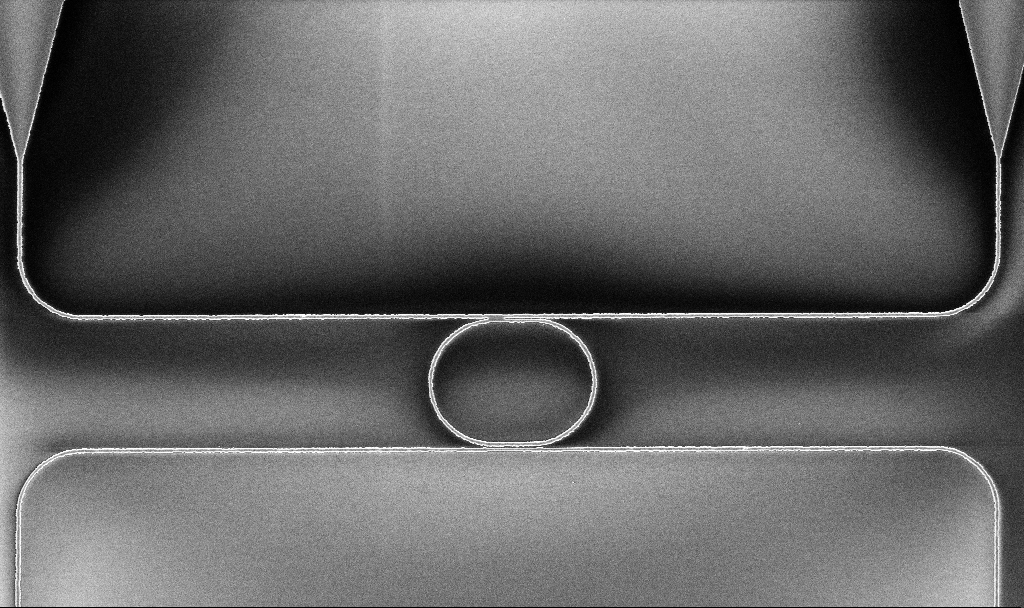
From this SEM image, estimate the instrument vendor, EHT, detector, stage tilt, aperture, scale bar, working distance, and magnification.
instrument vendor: Zeiss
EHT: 5 kV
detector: InLens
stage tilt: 0°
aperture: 30 µm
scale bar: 10000 nm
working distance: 10.1 mm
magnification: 2.38 K X